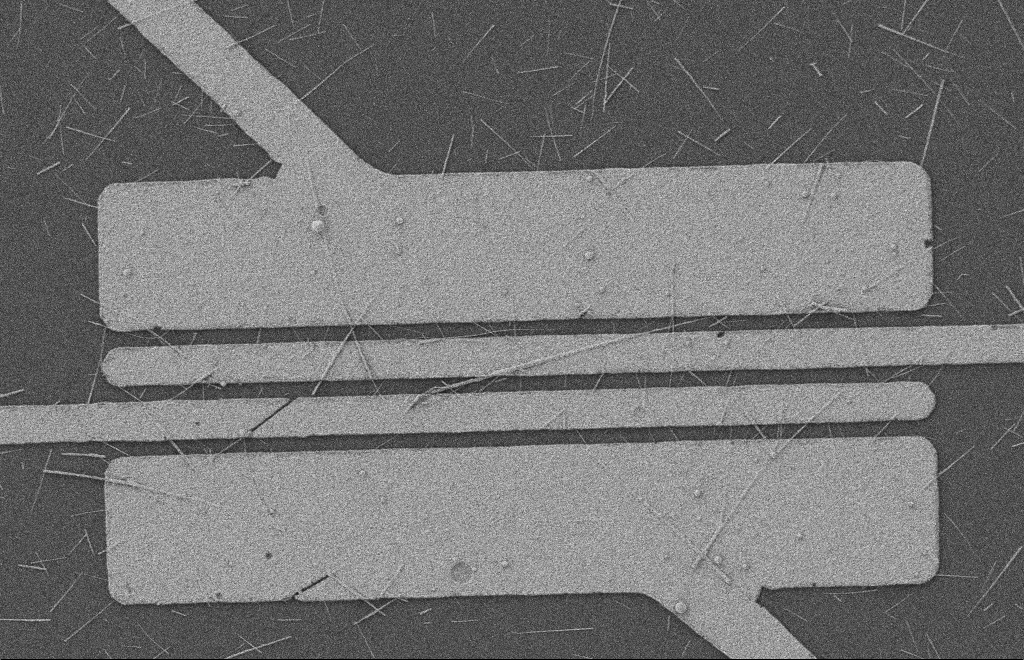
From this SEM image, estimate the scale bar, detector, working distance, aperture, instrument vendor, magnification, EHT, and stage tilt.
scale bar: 2000 nm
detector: SE2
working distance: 8 mm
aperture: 20 µm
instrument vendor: Zeiss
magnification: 5.01 K X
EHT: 2 kV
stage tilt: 0°